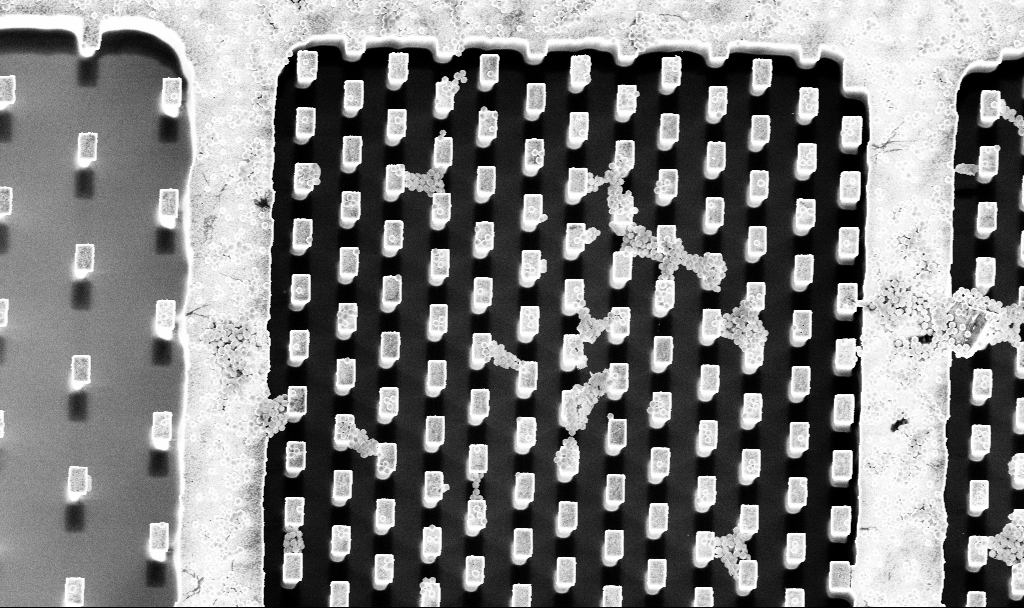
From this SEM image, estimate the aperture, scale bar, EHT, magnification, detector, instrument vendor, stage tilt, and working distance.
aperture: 30 µm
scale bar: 10000 nm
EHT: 5 kV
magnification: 4.24 K X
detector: InLens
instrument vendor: Zeiss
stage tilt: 5°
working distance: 3.3 mm